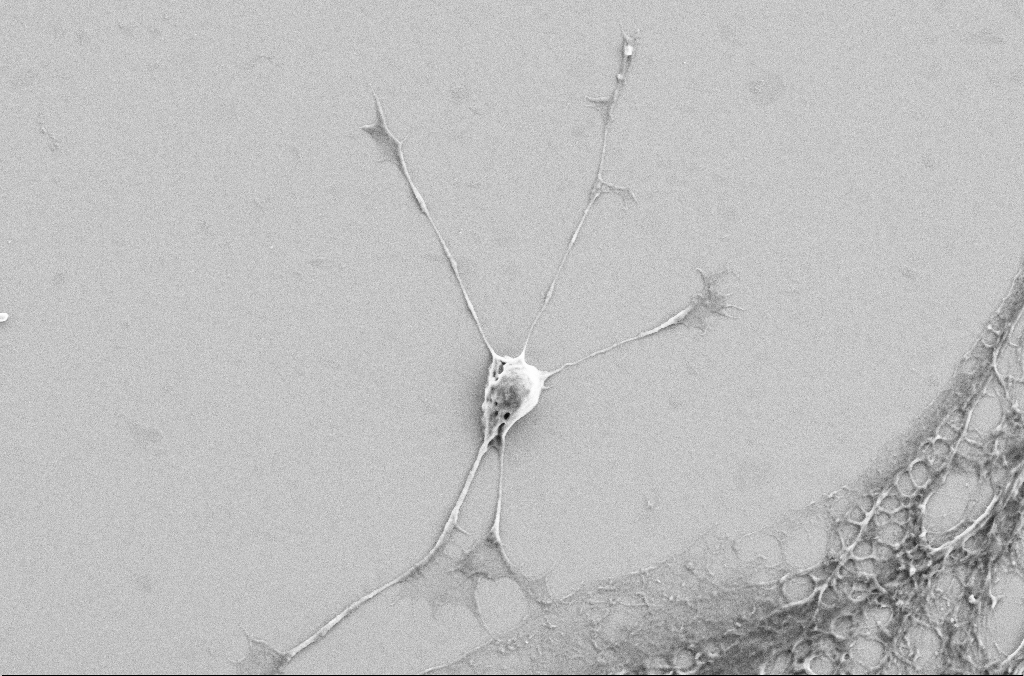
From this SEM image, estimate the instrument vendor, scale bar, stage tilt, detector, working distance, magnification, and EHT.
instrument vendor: Zeiss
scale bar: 10000 nm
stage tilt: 0°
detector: SE2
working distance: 3.2 mm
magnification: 4 K X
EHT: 5 kV